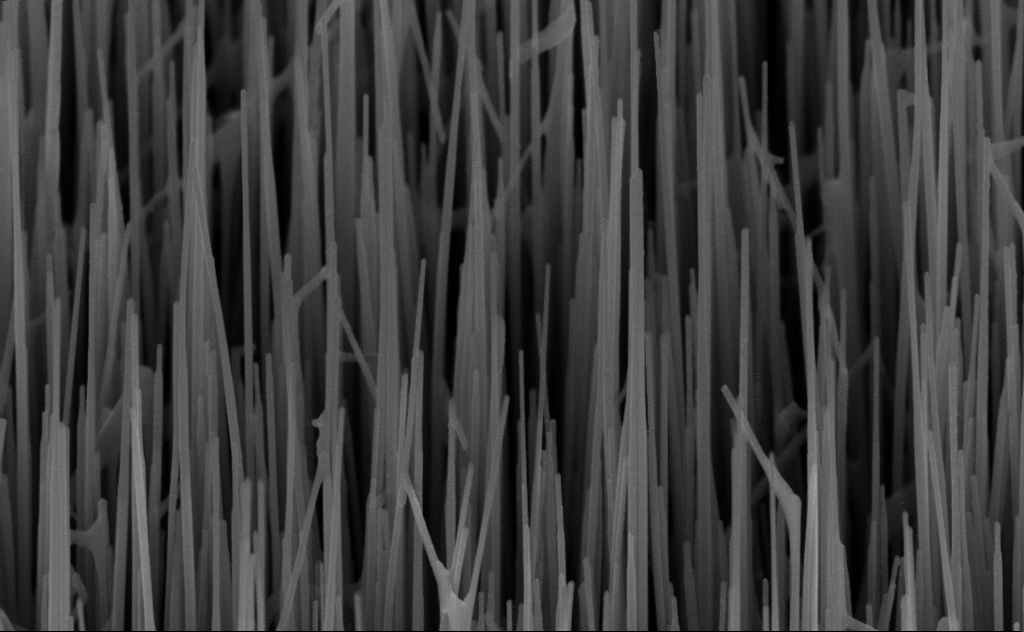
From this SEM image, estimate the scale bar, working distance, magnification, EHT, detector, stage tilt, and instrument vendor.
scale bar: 200 nm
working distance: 6 mm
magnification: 80 K X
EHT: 10 kV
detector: InLens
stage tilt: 45°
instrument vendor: Zeiss